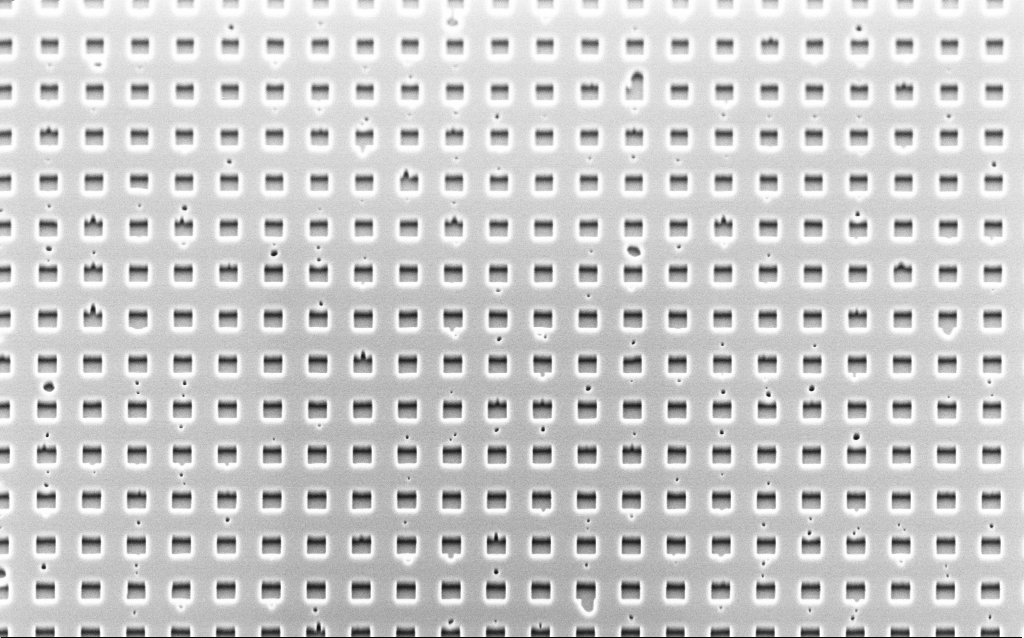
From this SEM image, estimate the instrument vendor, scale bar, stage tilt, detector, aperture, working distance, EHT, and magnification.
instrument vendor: Zeiss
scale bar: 2000 nm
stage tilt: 45°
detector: InLens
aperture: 30 µm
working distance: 3.3 mm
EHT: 2 kV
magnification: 33.11 K X